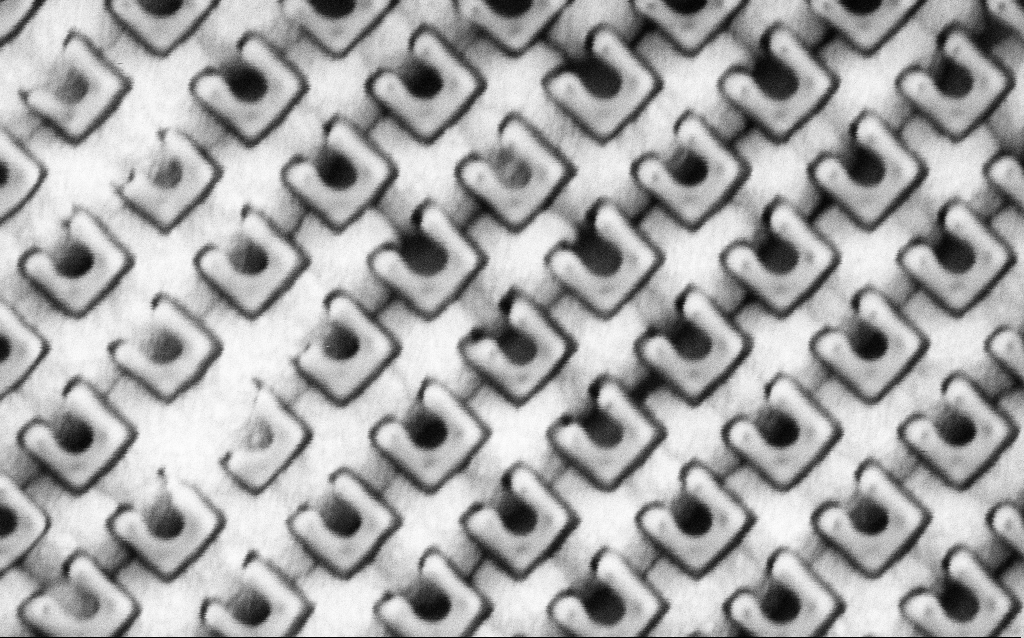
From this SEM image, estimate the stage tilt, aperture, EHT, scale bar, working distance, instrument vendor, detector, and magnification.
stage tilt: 0°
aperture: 30 µm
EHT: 1.5 kV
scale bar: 200 nm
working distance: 8.1 mm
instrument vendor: Zeiss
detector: SE2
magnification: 97.97 K X